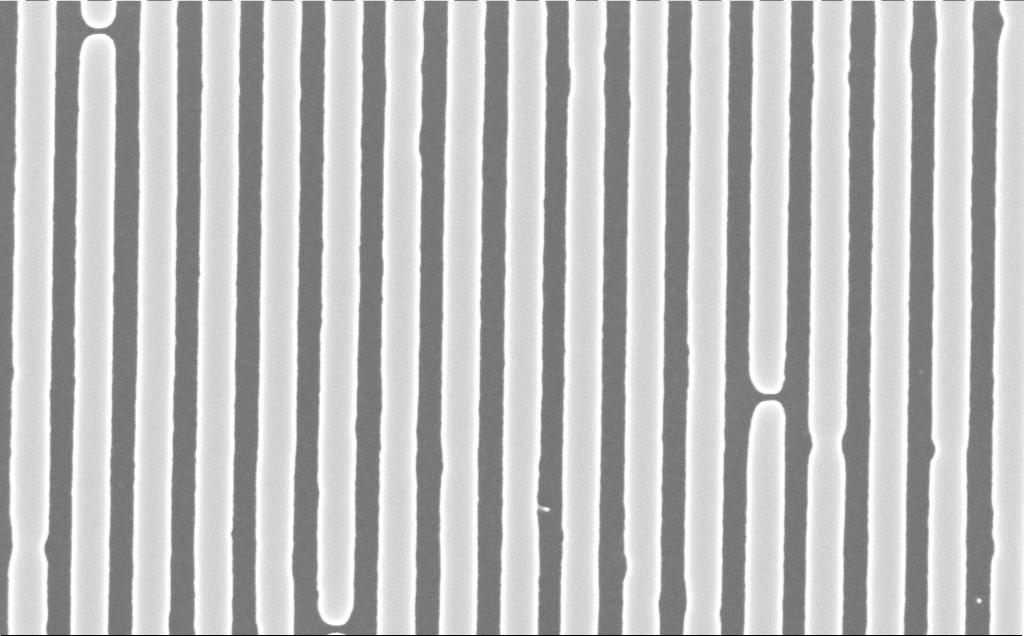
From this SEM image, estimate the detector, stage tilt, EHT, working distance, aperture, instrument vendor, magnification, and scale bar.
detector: InLens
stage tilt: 0°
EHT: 10 kV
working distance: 6 mm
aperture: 30 µm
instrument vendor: Zeiss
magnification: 75 K X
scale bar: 200 nm